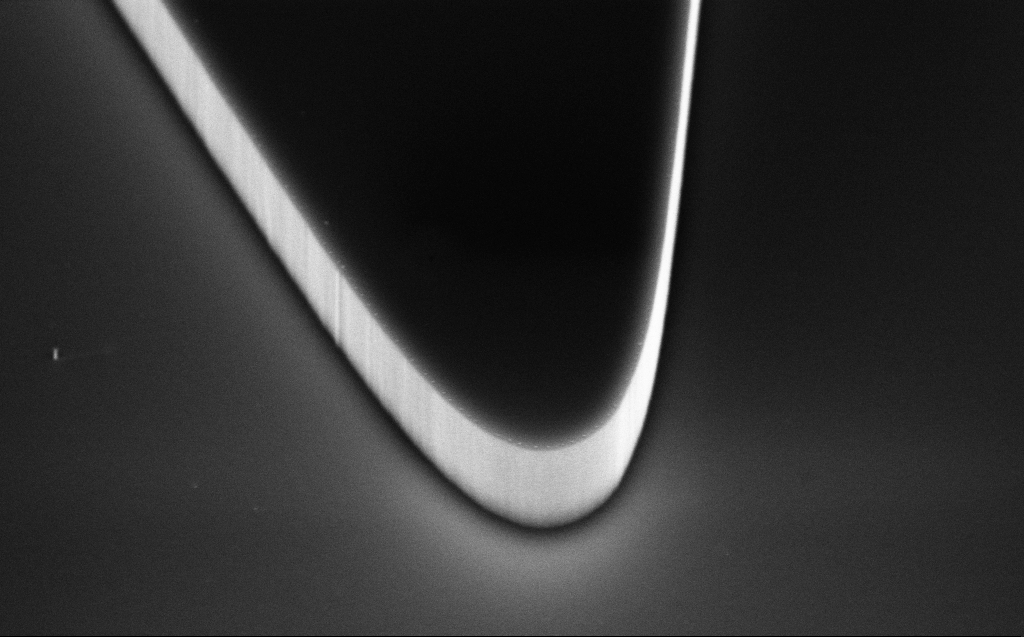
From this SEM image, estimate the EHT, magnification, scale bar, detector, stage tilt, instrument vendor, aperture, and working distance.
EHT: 5 kV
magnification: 39.42 K X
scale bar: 1000 nm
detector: InLens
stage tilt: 45°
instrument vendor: Zeiss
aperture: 30 µm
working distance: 5 mm